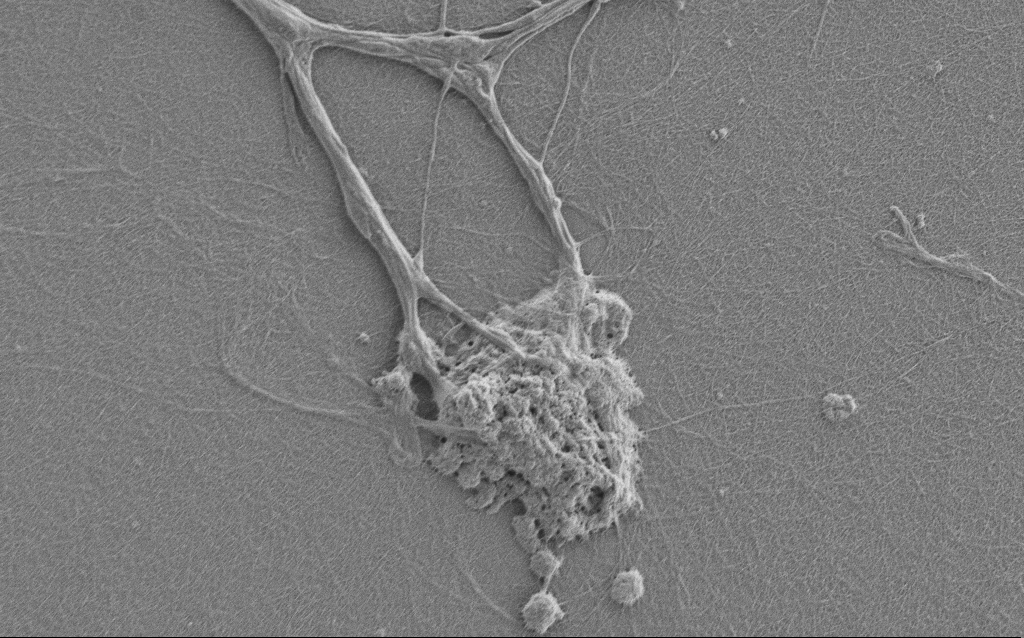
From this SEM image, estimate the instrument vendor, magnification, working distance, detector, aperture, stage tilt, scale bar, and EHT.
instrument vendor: Zeiss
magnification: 7.5 K X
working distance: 6 mm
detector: SE2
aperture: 30 µm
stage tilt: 0°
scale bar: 2000 nm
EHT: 1 kV